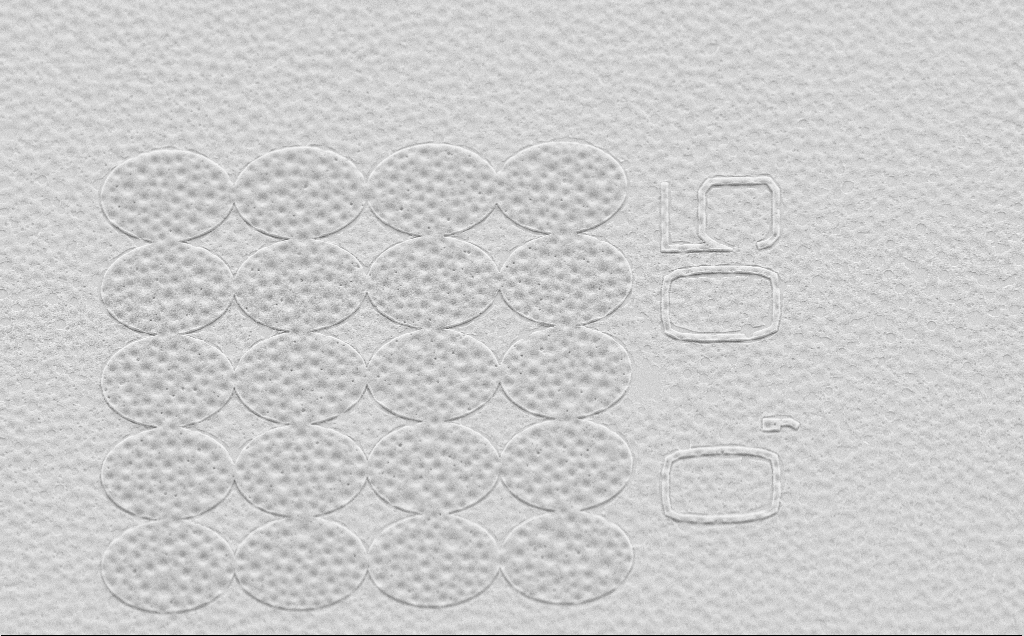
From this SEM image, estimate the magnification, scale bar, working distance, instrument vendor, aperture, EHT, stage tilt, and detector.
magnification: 4.92 K X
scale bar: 10000 nm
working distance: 6 mm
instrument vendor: Zeiss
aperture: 30 µm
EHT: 5 kV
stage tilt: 45°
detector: SE2